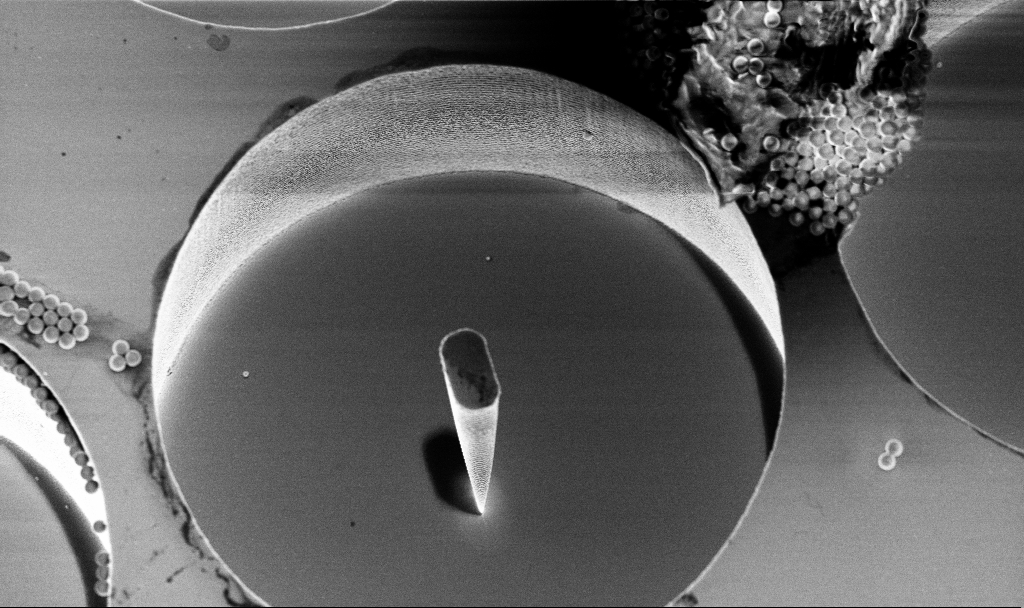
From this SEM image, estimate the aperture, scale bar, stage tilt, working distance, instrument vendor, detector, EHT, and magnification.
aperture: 30 µm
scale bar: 2000 nm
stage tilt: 15°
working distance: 4.7 mm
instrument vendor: Zeiss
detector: InLens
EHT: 4 kV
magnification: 7.83 K X